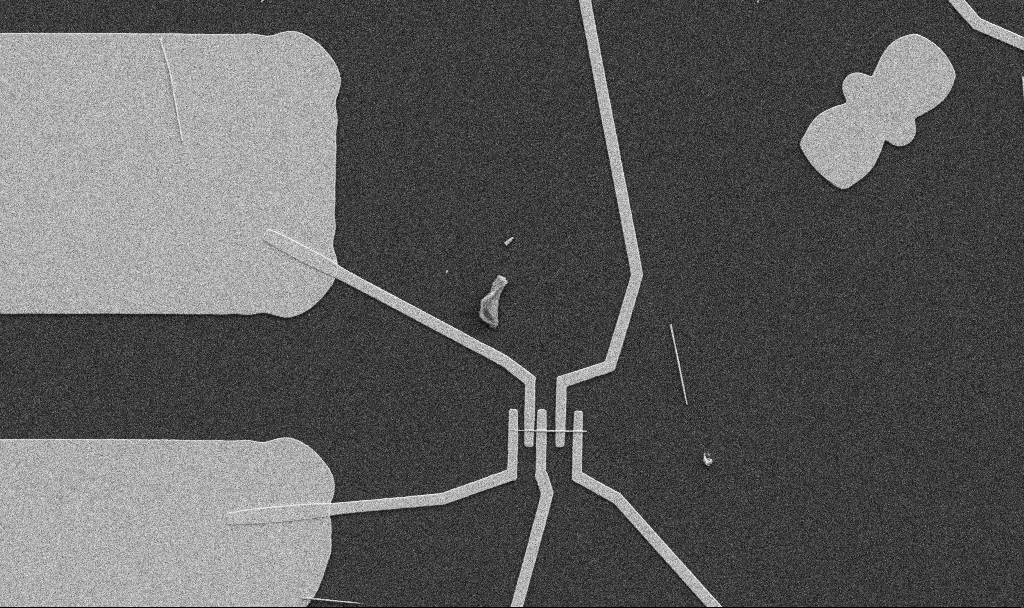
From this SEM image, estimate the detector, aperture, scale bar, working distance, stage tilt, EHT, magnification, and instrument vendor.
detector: SE2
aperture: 30 µm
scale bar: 10000 nm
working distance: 10.7 mm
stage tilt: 0°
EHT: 5 kV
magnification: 5 K X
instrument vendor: Zeiss